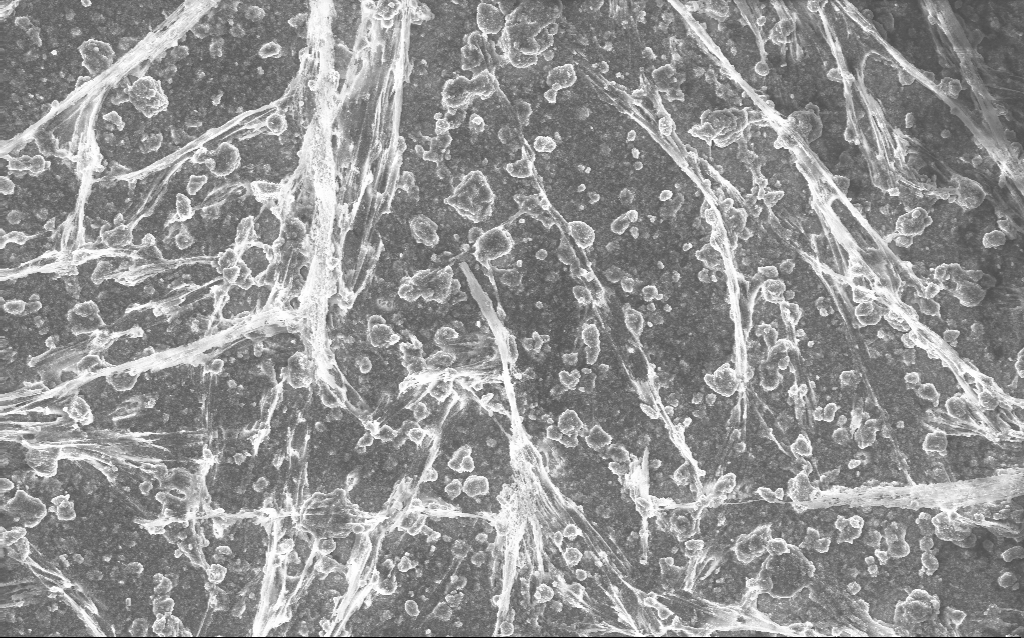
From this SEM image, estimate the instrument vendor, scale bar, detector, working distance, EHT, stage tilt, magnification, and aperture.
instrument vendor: Zeiss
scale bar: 10000 nm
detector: InLens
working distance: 2.8 mm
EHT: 10 kV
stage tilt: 0°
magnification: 1.69 K X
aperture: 30 µm